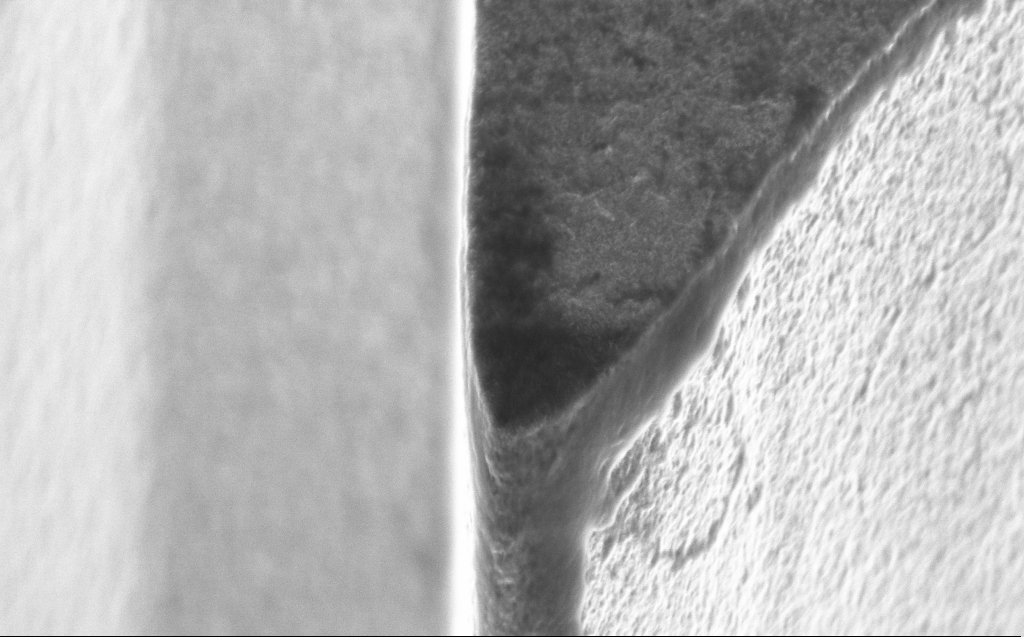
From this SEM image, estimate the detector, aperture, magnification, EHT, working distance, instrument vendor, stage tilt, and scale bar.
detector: InLens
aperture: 30 µm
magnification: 51.05 K X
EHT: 5 kV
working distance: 5 mm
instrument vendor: Zeiss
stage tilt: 45°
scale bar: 1000 nm